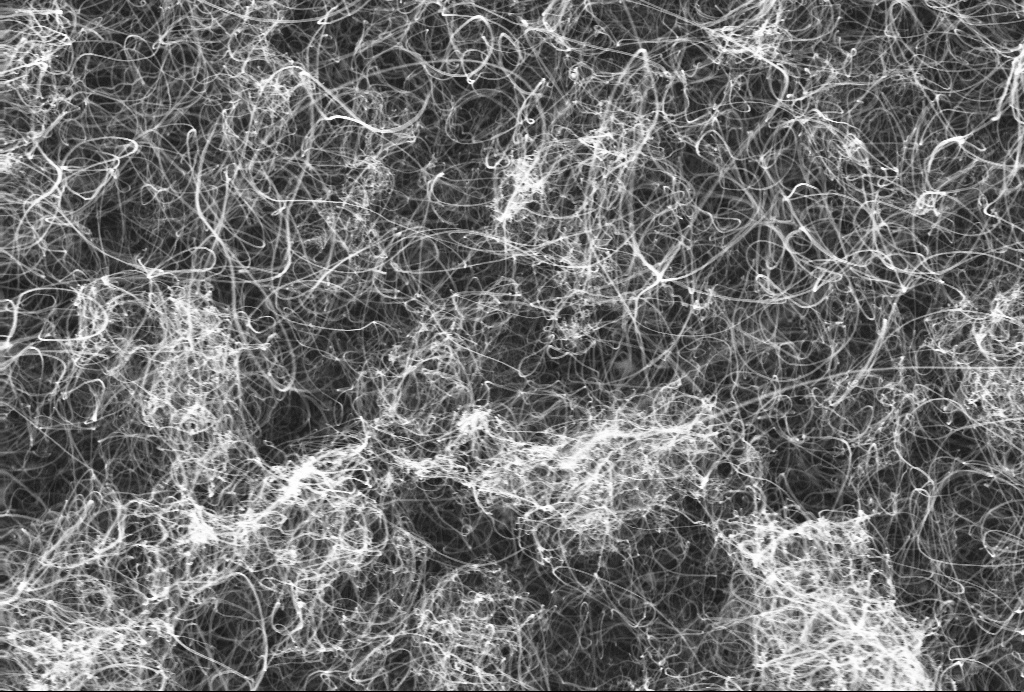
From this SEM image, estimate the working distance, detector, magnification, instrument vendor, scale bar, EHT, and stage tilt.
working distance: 3.1 mm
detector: InLens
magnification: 36.88 K X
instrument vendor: Zeiss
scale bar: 1000 nm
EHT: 10 kV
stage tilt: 0°